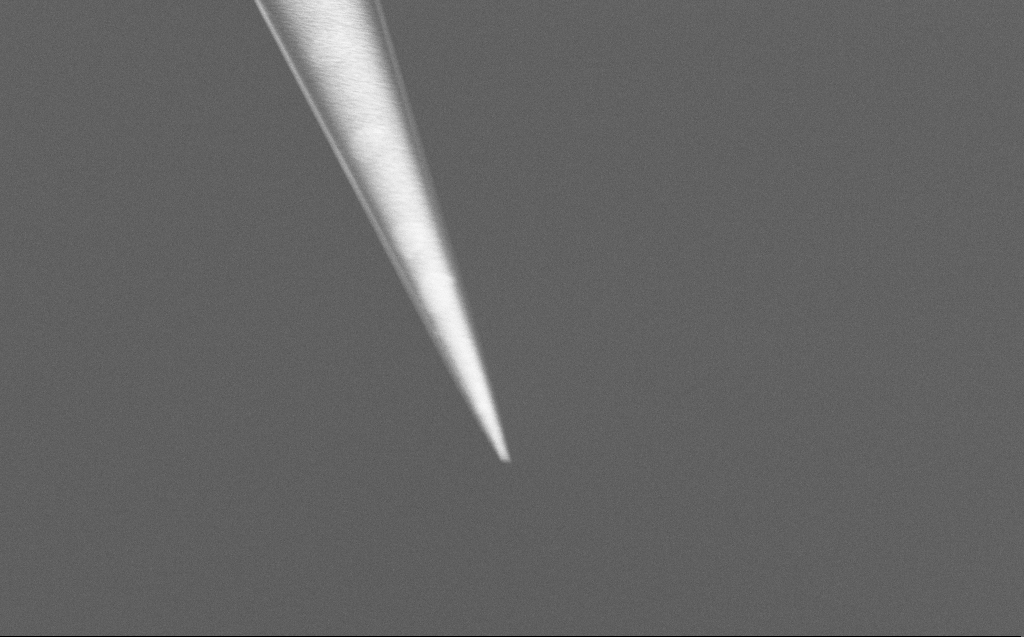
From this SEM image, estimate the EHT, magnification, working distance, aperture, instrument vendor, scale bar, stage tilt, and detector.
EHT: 5 kV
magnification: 5 K X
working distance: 7 mm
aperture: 30 µm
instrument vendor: Zeiss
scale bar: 10000 nm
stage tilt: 45°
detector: InLens